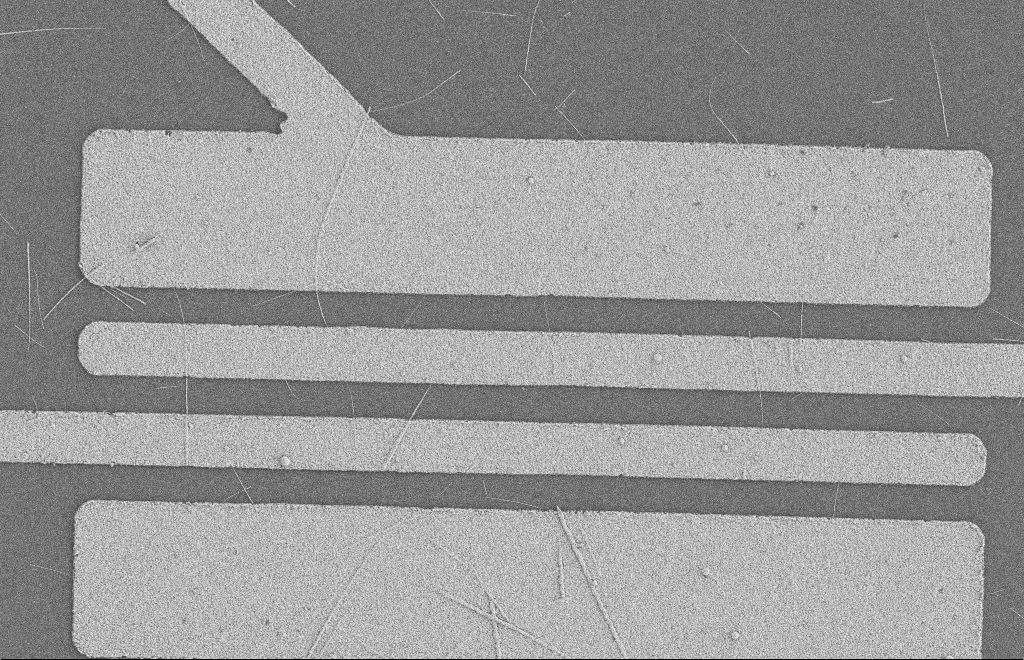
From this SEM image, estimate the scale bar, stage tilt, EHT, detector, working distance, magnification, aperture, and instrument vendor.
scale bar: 2000 nm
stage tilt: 0°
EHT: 2 kV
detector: SE2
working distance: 8 mm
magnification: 5.46 K X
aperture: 20 µm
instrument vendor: Zeiss